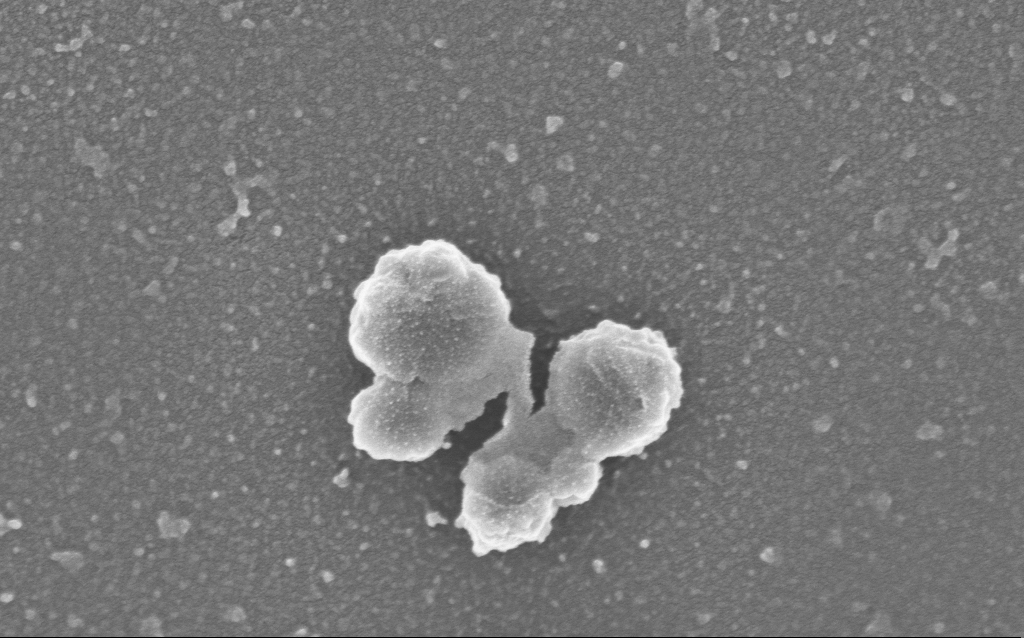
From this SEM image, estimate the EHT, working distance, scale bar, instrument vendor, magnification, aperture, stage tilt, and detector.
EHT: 3 kV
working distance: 3.1 mm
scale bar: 200 nm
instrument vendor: Zeiss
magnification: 100 K X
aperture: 30 µm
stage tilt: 0°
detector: InLens